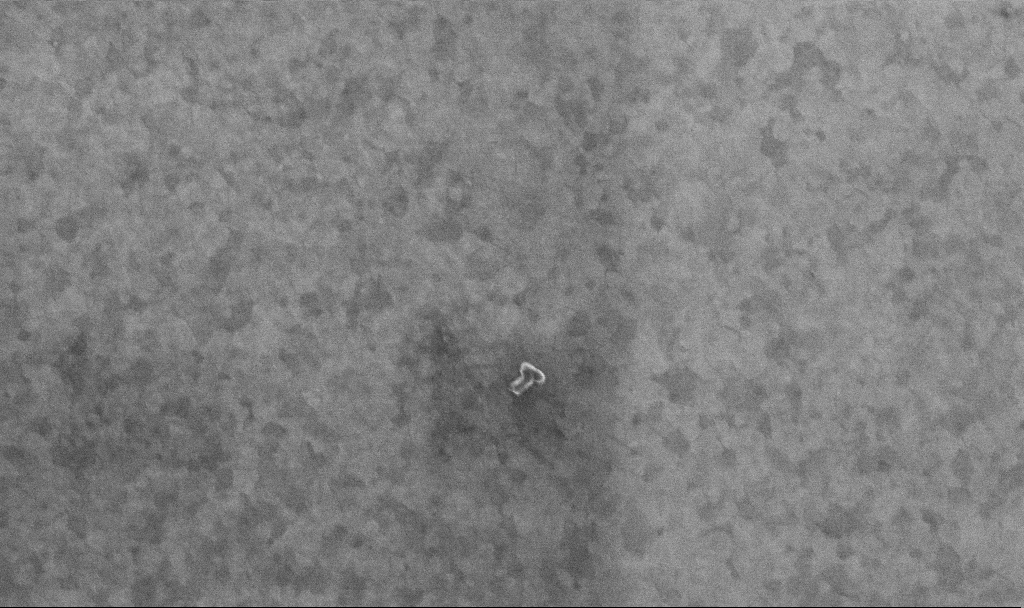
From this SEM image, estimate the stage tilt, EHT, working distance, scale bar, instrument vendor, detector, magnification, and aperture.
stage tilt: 0°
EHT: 10 kV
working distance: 3.4 mm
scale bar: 200 nm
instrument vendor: Zeiss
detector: InLens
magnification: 99.94 K X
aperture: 30 µm